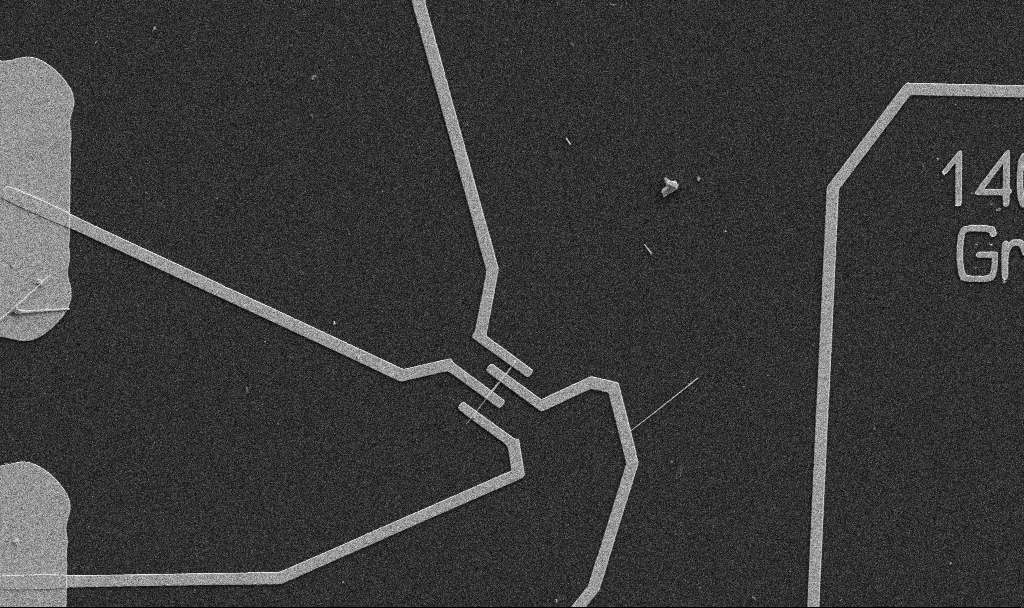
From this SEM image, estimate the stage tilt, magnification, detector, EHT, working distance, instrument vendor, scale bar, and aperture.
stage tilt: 0°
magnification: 5 K X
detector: SE2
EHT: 5 kV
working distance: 10.7 mm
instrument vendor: Zeiss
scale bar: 10000 nm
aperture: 30 µm